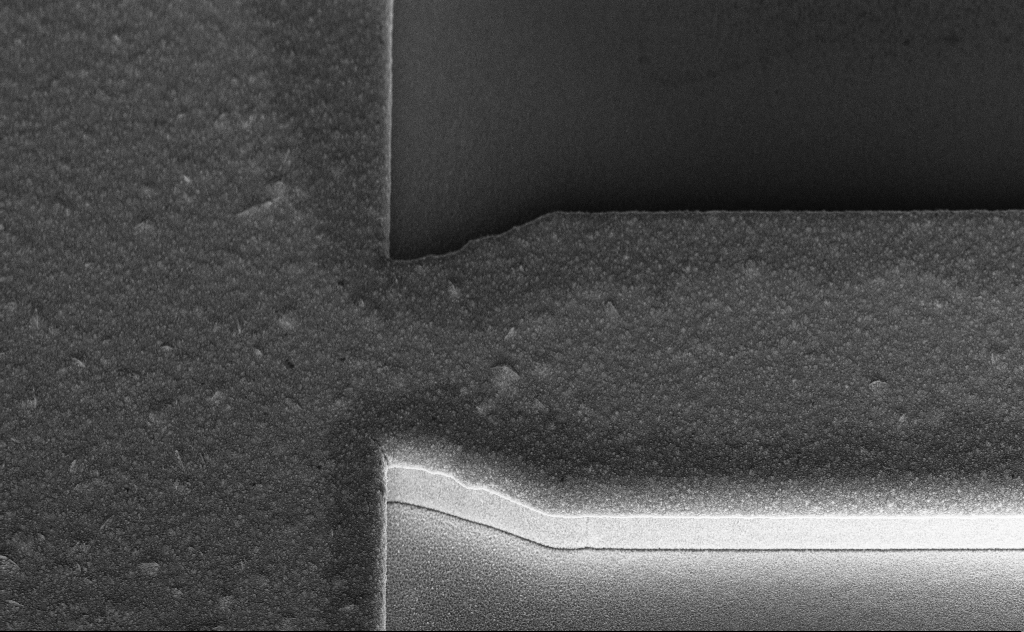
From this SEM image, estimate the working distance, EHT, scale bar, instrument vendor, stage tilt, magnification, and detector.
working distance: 11 mm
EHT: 10 kV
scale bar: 2000 nm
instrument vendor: Zeiss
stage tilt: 45°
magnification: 9.21 K X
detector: InLens